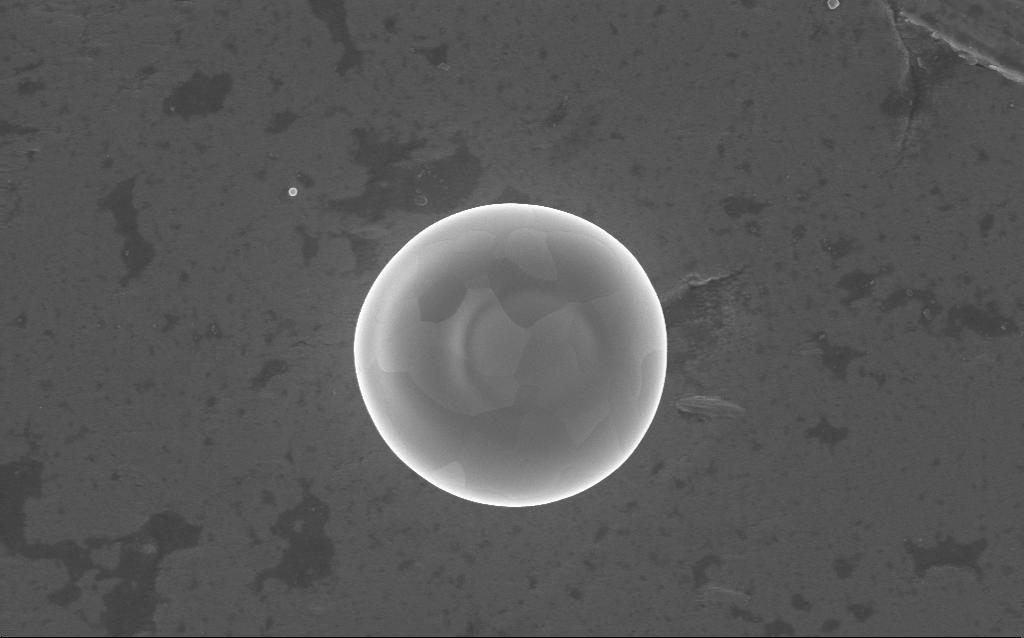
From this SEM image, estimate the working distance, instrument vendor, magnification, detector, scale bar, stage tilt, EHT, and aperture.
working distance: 4 mm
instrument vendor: Zeiss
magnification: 34 K X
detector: InLens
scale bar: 2000 nm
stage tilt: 0°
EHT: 5 kV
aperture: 30 µm